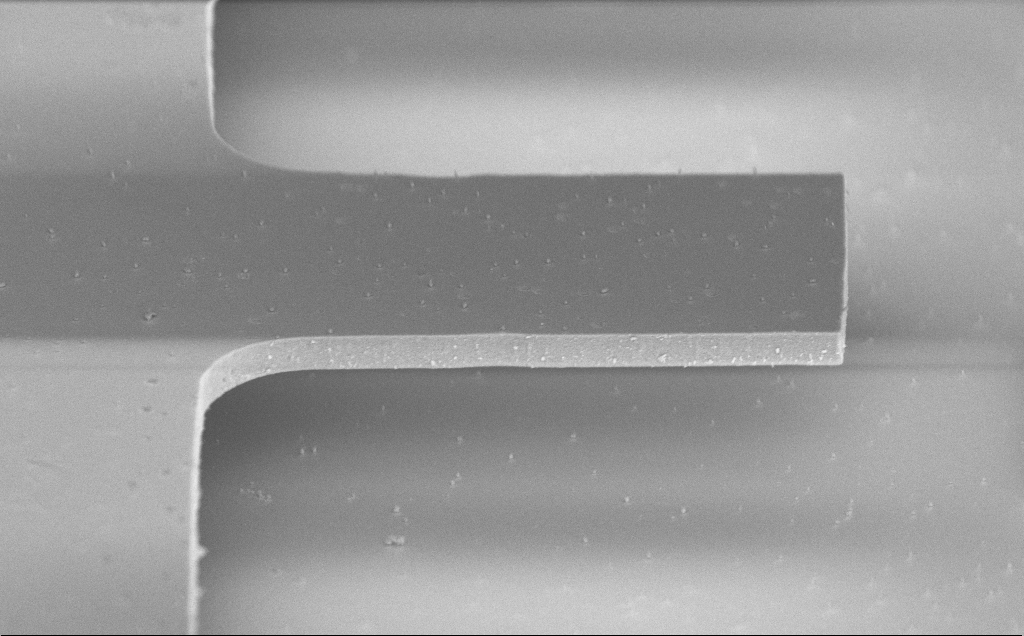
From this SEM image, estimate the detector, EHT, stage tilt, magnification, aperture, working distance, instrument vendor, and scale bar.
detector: InLens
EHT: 3 kV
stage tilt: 70°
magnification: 9.45 K X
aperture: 30 µm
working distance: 5 mm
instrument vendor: Zeiss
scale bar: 2000 nm